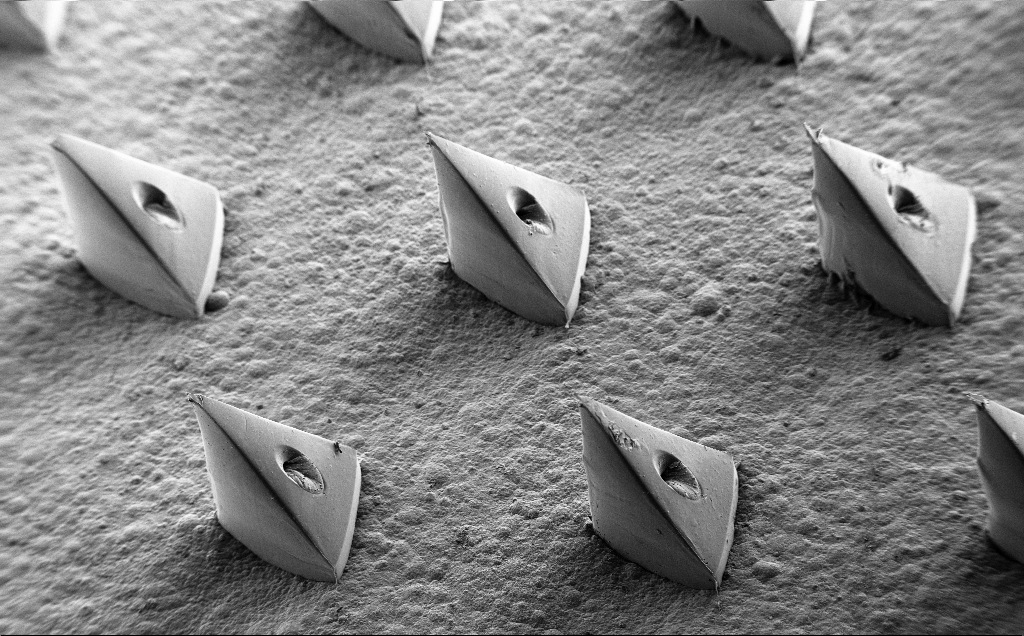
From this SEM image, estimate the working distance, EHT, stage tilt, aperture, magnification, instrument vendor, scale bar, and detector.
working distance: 12 mm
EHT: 5 kV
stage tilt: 35°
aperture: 30 µm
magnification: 0.096 K X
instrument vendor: Zeiss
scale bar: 200000 nm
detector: SE2